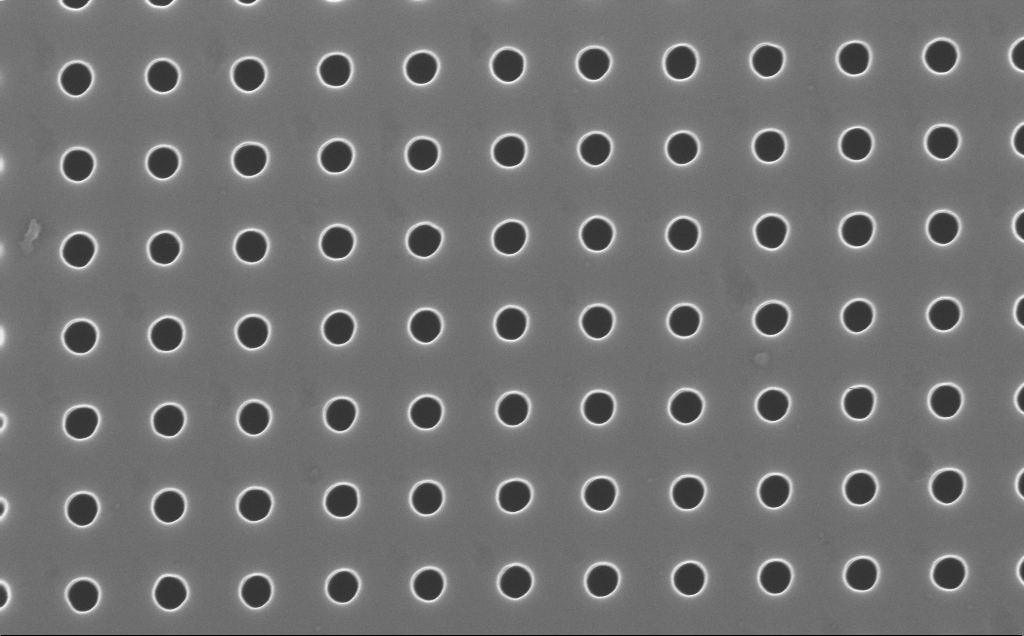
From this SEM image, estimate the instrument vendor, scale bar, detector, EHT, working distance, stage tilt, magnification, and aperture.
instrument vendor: Zeiss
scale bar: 200 nm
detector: InLens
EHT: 10 kV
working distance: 4 mm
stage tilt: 0°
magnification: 106.33 K X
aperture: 30 µm